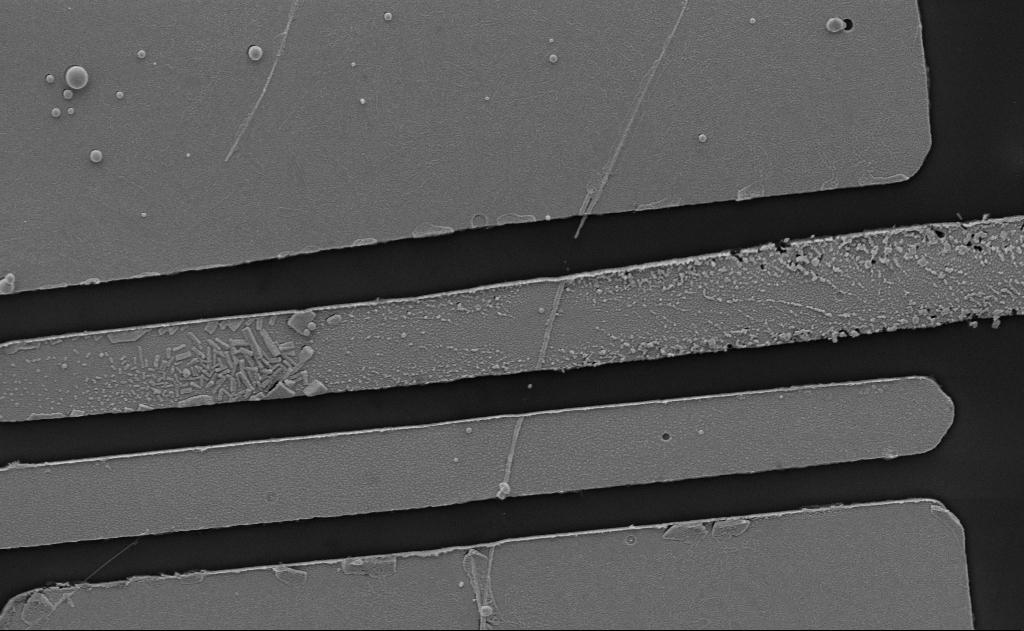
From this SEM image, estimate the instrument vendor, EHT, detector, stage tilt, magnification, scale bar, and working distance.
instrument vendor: Zeiss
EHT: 5 kV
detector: SE2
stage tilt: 0°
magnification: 11.7 K X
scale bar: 2000 nm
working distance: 15 mm